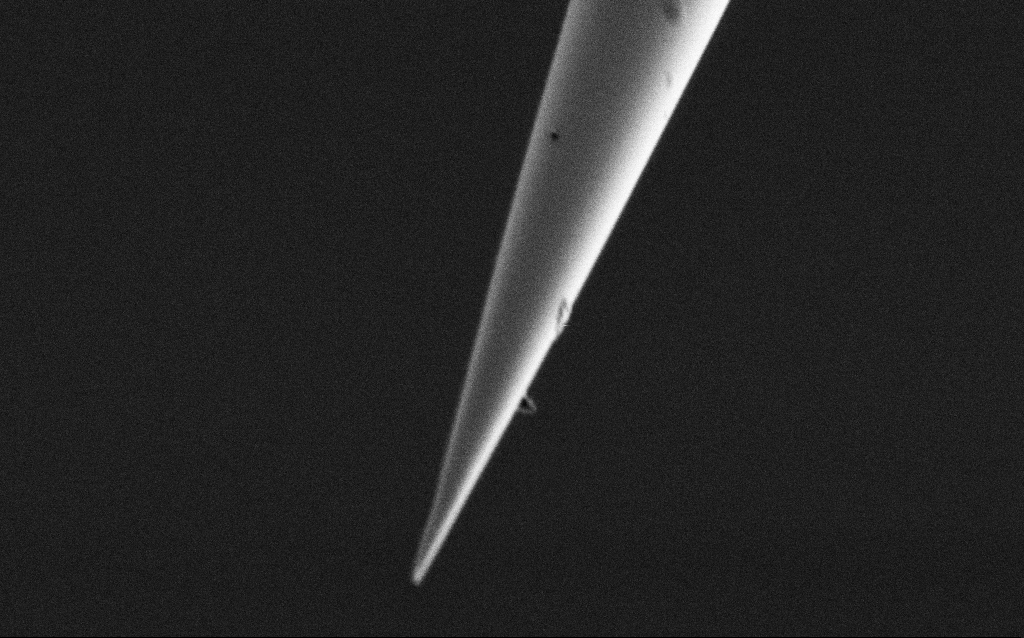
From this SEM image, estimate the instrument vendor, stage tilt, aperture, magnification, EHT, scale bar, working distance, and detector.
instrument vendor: Zeiss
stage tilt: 45°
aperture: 30 µm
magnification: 25 K X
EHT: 1 kV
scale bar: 2000 nm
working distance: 7.4 mm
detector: SE2